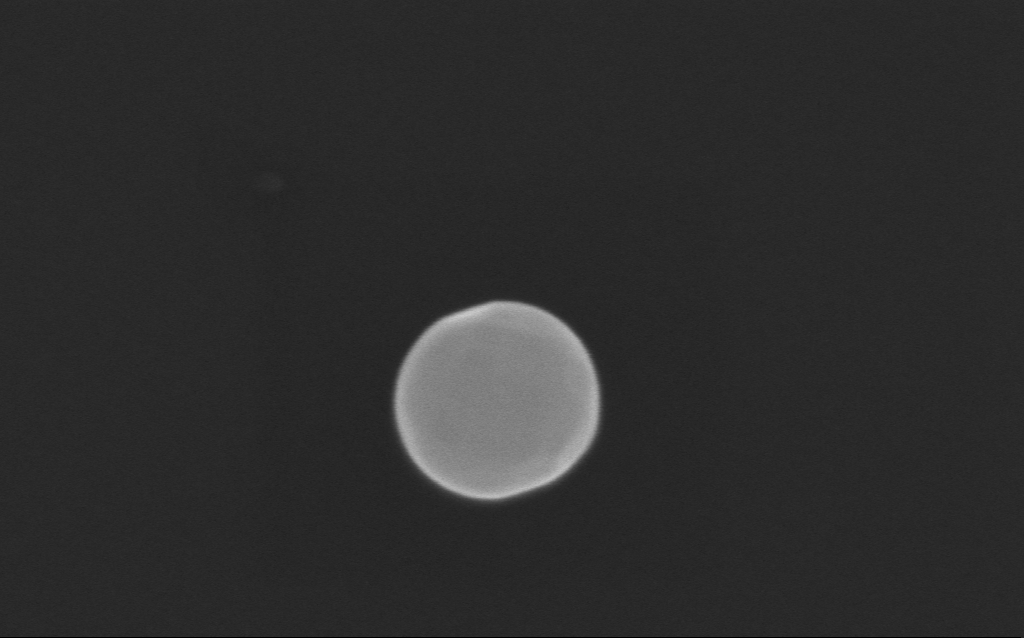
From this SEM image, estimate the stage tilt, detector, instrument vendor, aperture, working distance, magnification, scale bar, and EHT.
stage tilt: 0°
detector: InLens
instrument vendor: Zeiss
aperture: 30 µm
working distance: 3 mm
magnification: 499.96 K X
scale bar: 100 nm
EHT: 10 kV